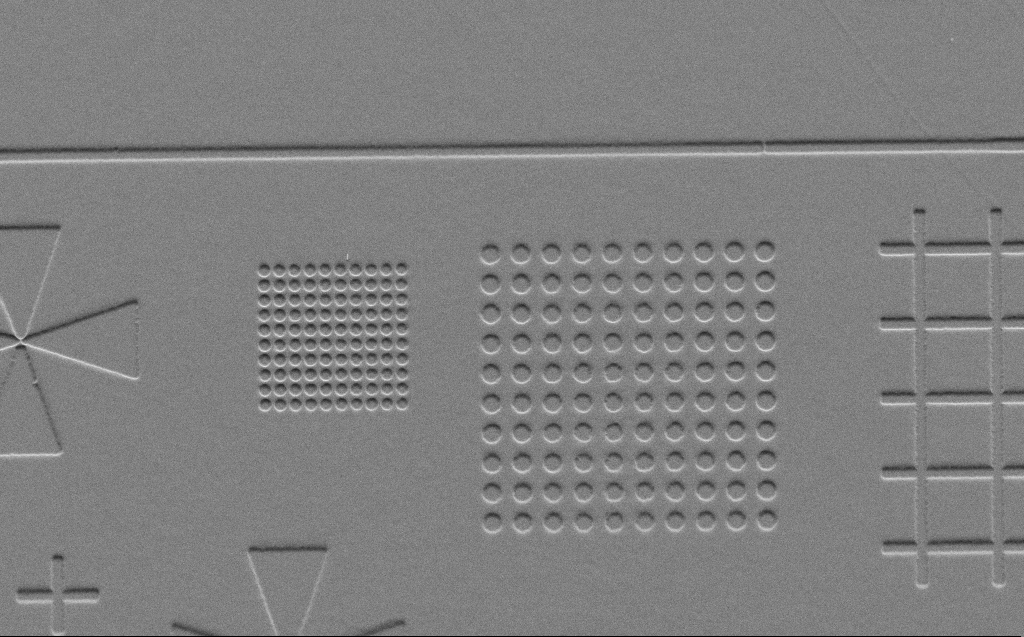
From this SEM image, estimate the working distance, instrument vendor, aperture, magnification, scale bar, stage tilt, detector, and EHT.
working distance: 5 mm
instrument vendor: Zeiss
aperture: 30 µm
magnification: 2.83 K X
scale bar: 10000 nm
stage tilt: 45°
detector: SE2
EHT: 3 kV